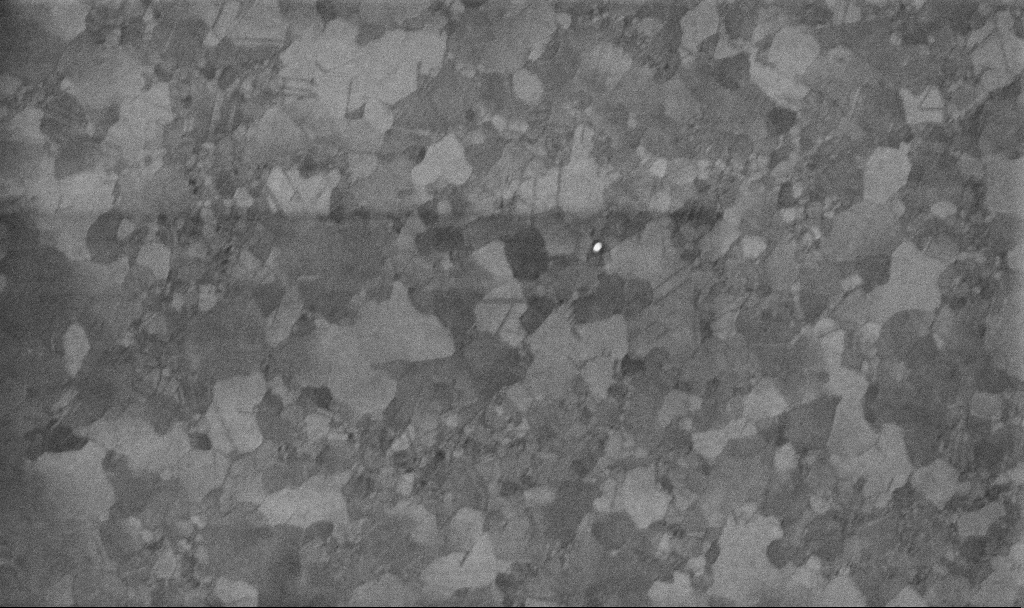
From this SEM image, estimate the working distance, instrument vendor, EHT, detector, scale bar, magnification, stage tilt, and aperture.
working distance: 3.4 mm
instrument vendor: Zeiss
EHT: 10 kV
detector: InLens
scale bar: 200 nm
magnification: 100 K X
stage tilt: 0°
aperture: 30 µm